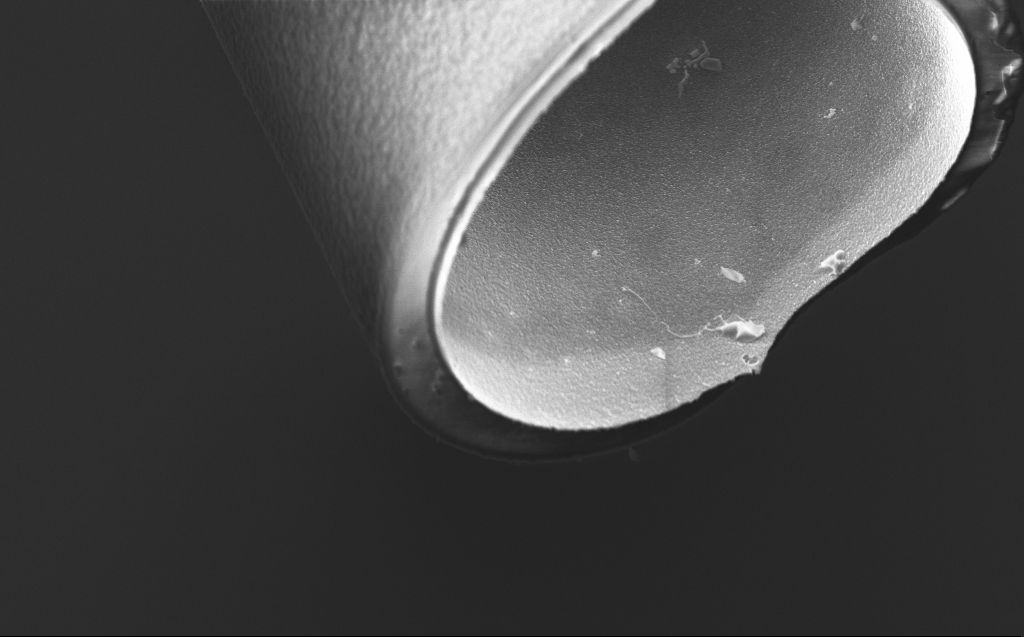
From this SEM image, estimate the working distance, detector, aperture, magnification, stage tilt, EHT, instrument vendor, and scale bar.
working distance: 4 mm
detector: InLens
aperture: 30 µm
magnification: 8.64 K X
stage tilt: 45°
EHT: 5 kV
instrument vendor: Zeiss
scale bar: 2000 nm